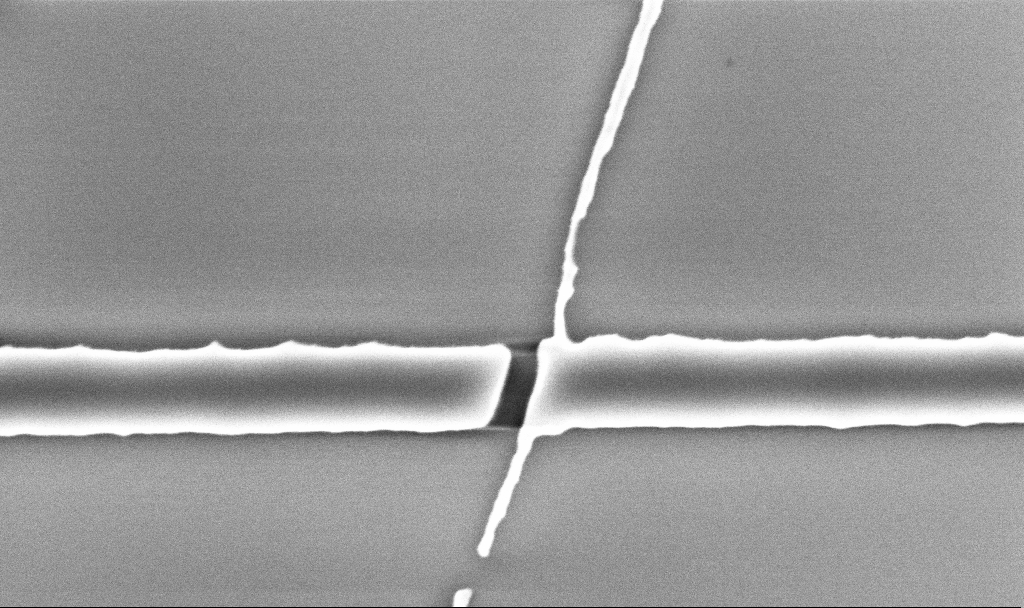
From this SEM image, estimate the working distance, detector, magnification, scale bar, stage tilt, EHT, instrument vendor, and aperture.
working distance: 5.2 mm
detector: InLens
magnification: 62.43 K X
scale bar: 1000 nm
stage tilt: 0°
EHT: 5 kV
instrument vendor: Zeiss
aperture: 30 µm